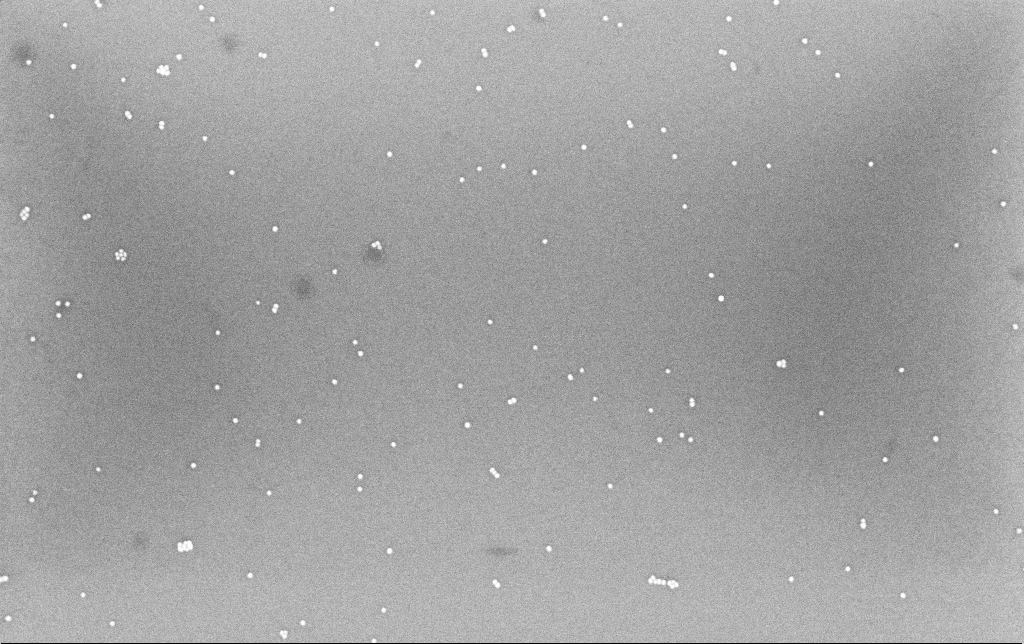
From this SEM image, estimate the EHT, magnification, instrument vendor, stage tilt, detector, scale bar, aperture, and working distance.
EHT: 8 kV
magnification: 100 K X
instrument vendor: Zeiss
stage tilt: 0°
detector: InLens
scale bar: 200 nm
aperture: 30 µm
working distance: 6.6 mm